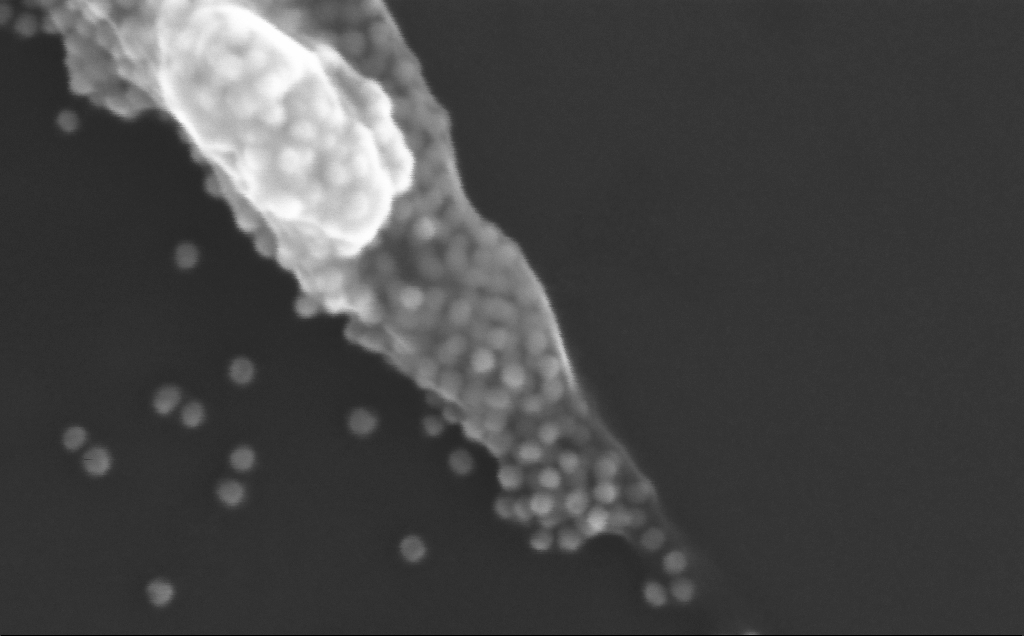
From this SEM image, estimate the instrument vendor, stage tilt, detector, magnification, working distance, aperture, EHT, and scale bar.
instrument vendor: Zeiss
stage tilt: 0°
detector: InLens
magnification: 581.65 K X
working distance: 3 mm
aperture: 30 µm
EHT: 10 kV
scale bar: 100 nm